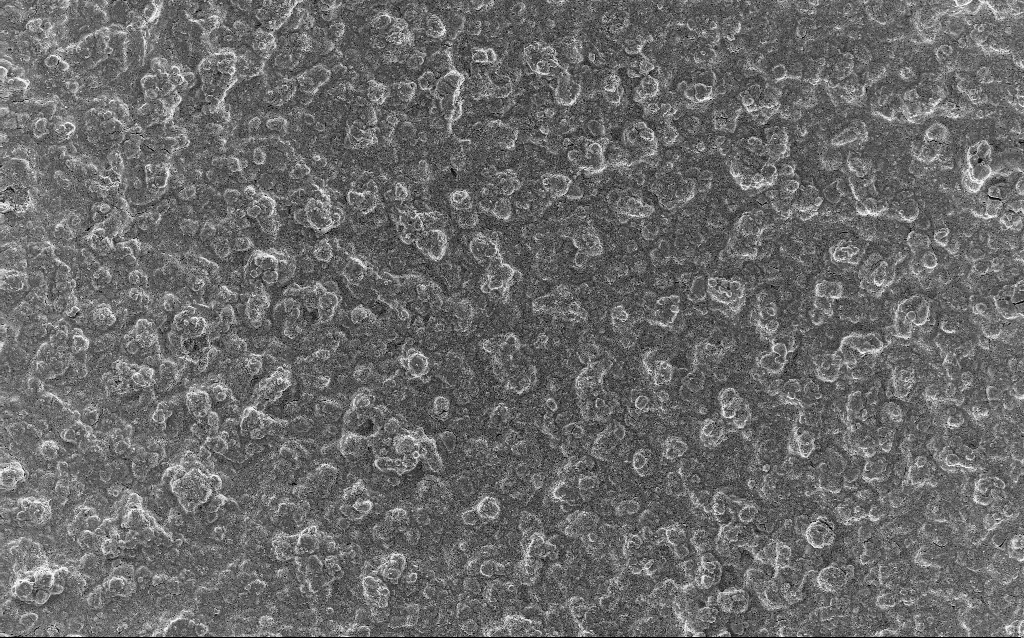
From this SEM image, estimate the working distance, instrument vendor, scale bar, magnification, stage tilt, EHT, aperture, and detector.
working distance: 3.4 mm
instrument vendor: Zeiss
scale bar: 20000 nm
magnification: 0.77 K X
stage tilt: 0°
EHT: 5 kV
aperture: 30 µm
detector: InLens